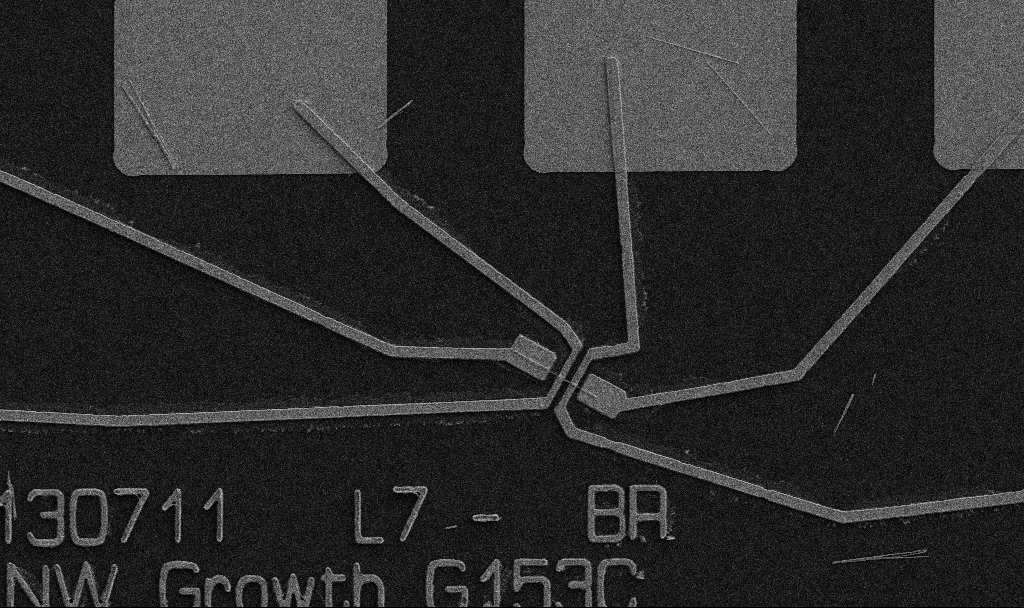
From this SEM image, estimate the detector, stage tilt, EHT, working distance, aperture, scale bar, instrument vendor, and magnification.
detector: SE2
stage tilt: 0°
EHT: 5 kV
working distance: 10.7 mm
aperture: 30 µm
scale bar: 10000 nm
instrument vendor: Zeiss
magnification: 5 K X